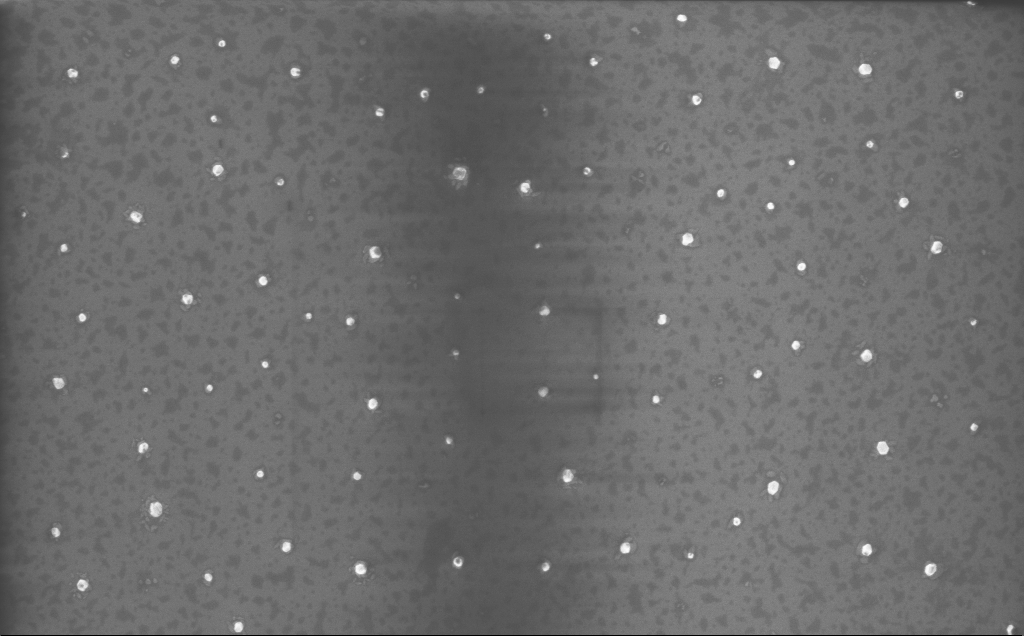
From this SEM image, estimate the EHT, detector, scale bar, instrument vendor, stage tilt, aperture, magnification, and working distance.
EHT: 10 kV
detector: InLens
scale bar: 1000 nm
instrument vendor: Zeiss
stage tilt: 0°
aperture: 30 µm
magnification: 23.13 K X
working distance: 4 mm